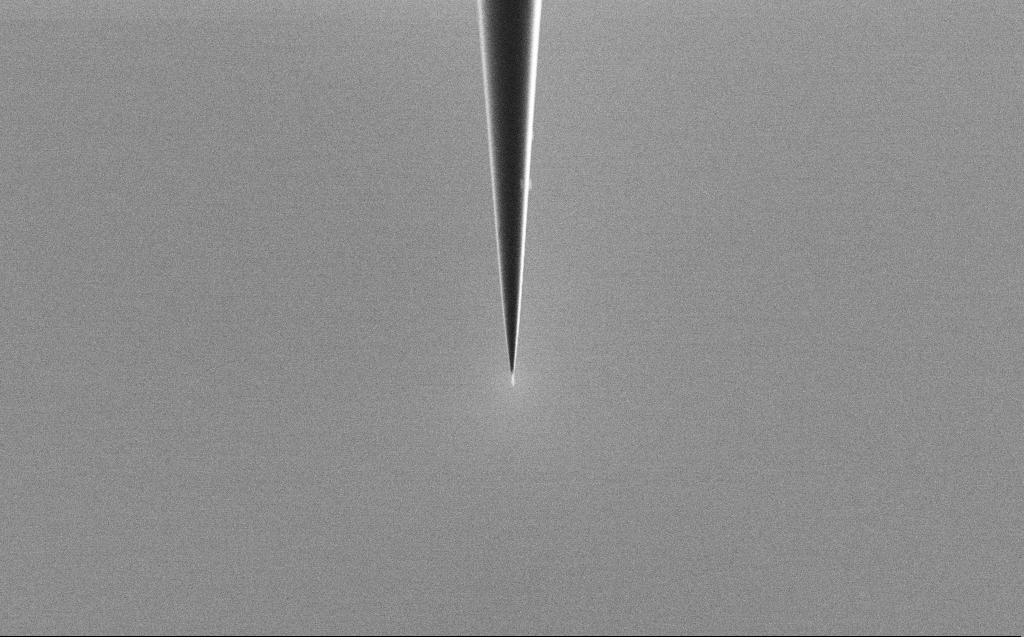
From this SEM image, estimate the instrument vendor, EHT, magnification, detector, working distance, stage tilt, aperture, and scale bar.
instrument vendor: Zeiss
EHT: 2 kV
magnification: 5 K X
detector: SE2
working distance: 6 mm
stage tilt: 45°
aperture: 30 µm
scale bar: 10000 nm